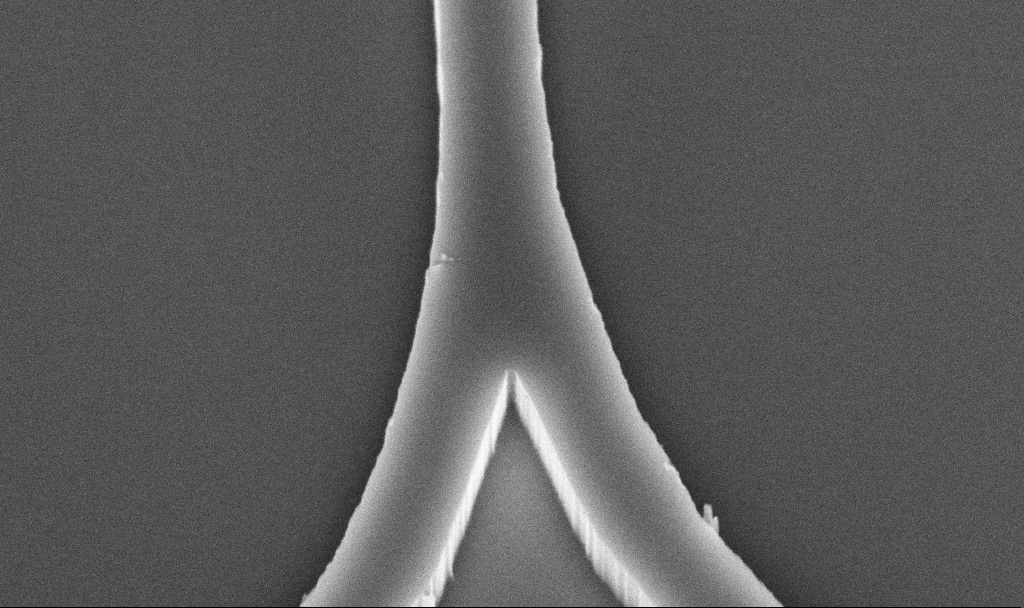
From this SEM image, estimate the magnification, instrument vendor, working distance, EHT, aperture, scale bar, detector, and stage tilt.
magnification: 71.22 K X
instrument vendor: Zeiss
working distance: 9.8 mm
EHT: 5 kV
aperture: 30 µm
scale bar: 1000 nm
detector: InLens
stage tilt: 45°